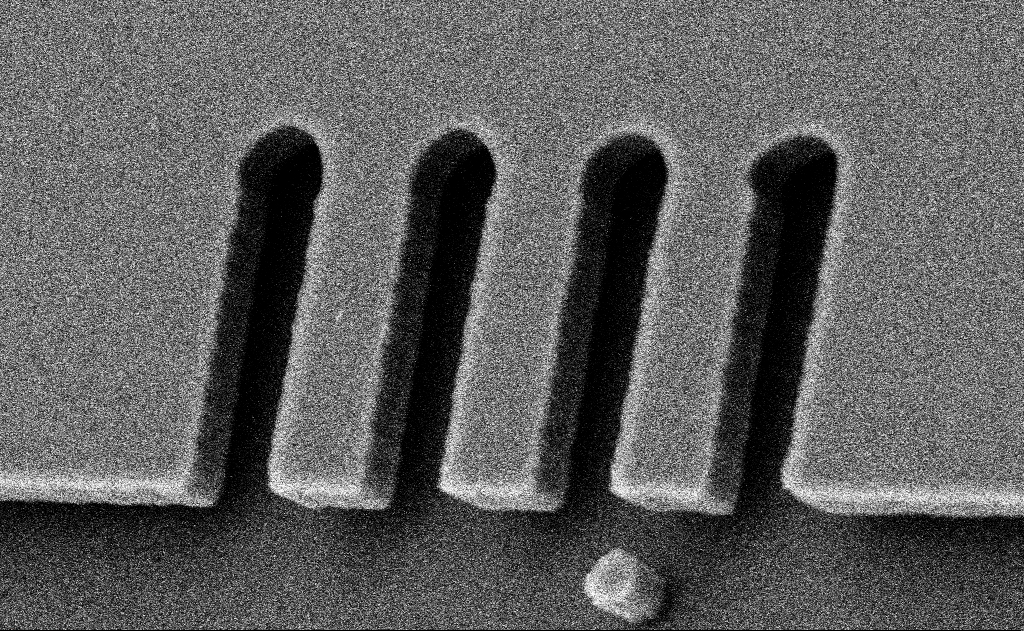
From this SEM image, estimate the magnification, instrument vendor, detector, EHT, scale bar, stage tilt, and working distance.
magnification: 9.2 K X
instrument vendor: Zeiss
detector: SE2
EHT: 10 kV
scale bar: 2000 nm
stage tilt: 35°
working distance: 10 mm